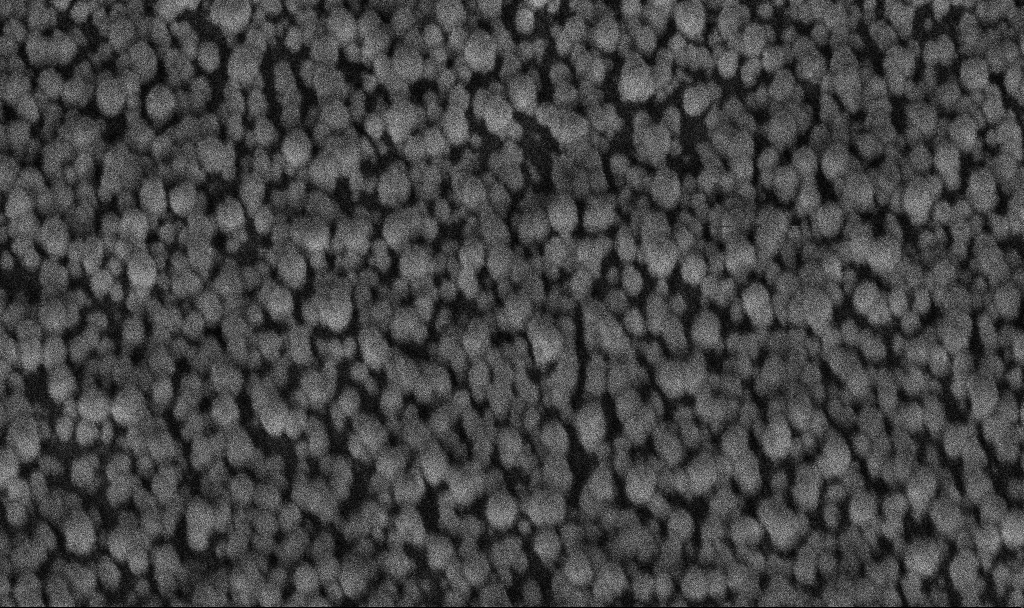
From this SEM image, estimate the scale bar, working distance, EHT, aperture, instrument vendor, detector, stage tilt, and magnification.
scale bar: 200 nm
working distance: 6.4 mm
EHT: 5 kV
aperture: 30 µm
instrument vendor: Zeiss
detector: InLens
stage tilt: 45°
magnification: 176.19 K X